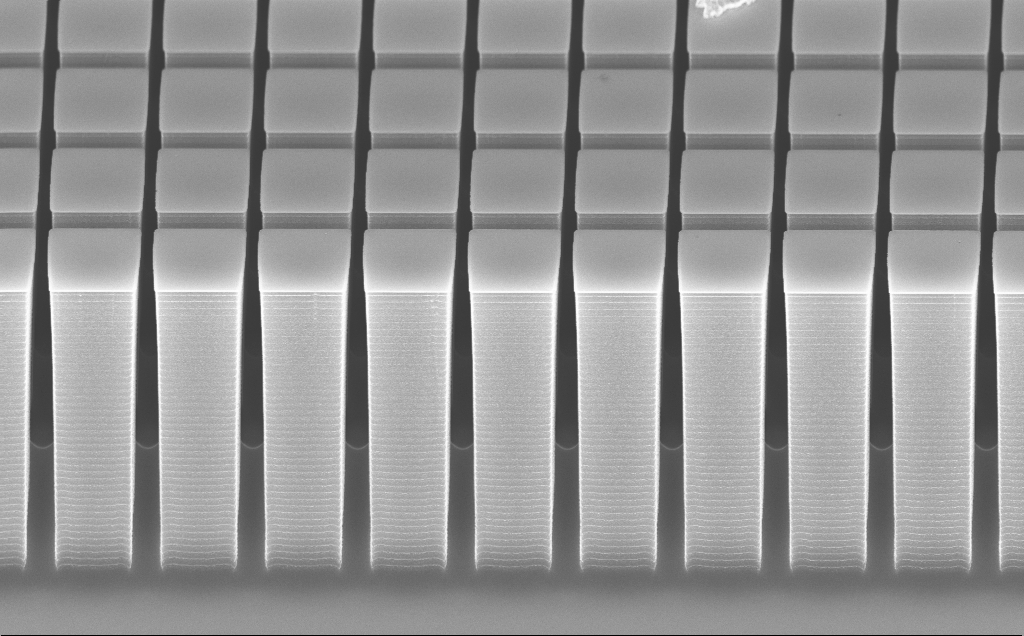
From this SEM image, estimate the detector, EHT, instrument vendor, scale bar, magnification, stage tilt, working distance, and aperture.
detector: InLens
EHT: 20 kV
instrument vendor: Zeiss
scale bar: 2000 nm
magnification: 7.68 K X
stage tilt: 60°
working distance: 7 mm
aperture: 30 µm